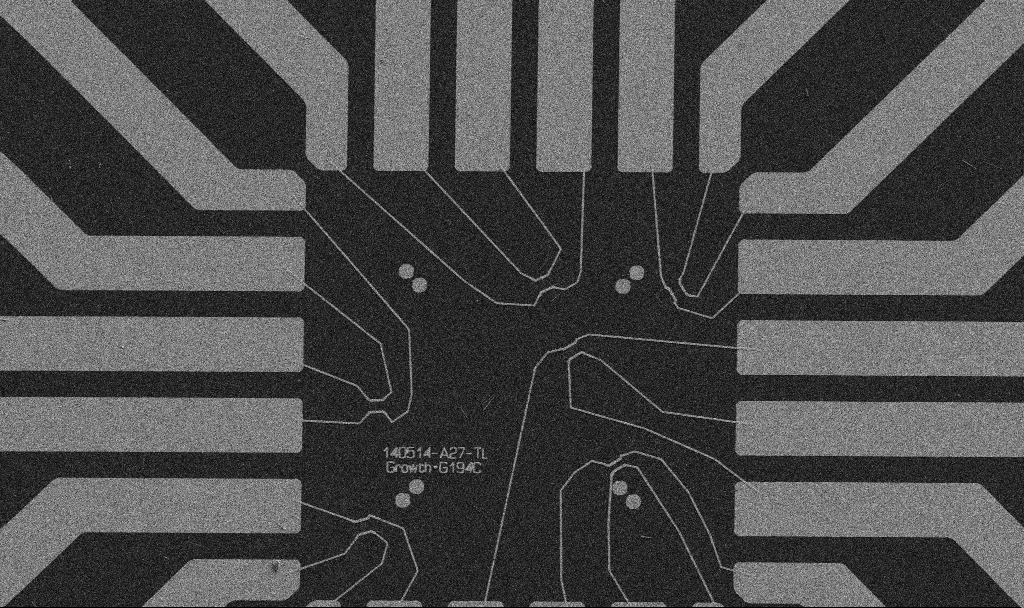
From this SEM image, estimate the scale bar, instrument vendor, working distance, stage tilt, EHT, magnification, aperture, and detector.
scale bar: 20000 nm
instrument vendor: Zeiss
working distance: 10.7 mm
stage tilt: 0°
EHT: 5 kV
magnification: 1 K X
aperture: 30 µm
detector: SE2